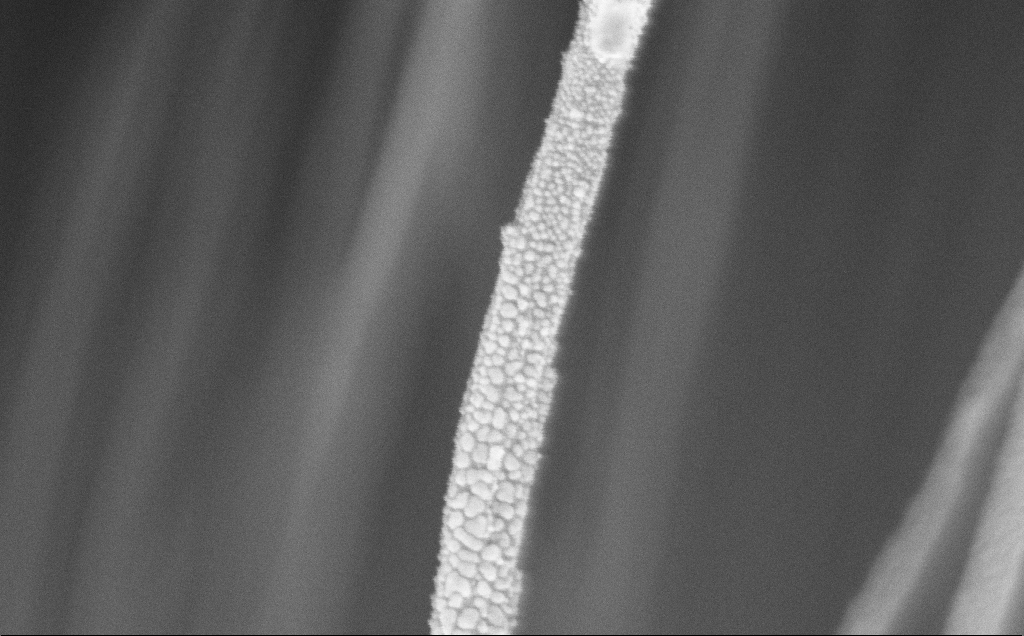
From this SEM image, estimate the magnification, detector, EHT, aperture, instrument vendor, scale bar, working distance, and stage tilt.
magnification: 163.96 K X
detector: InLens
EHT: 5 kV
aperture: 30 µm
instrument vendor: Zeiss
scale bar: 200 nm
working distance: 11 mm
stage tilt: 0°